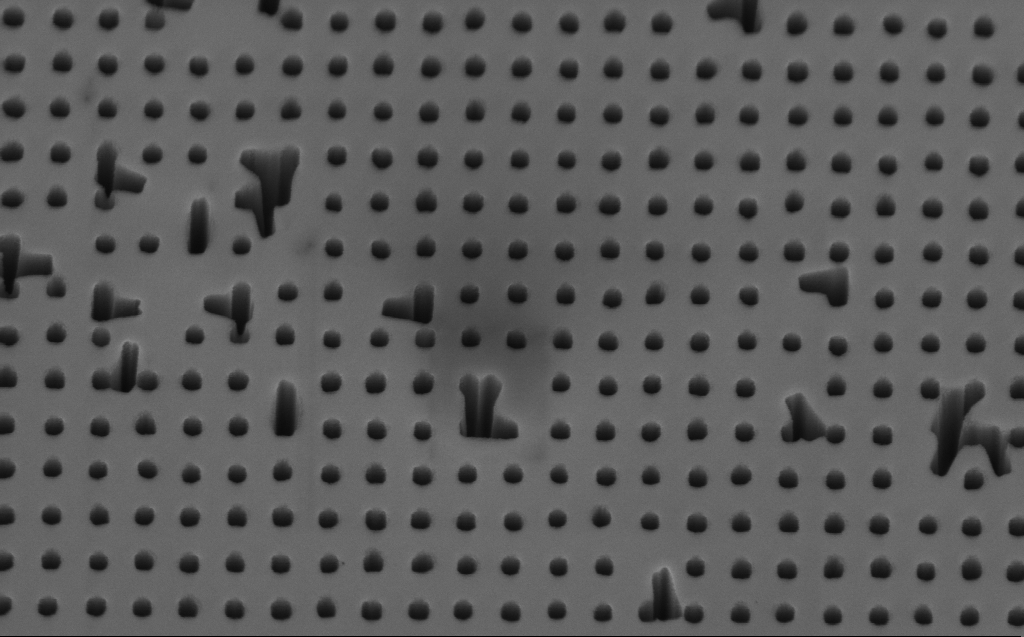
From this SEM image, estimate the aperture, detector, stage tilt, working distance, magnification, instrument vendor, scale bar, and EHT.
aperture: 30 µm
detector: InLens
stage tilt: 45°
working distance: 6 mm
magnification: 34.1 K X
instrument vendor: Zeiss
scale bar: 1000 nm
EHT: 3 kV